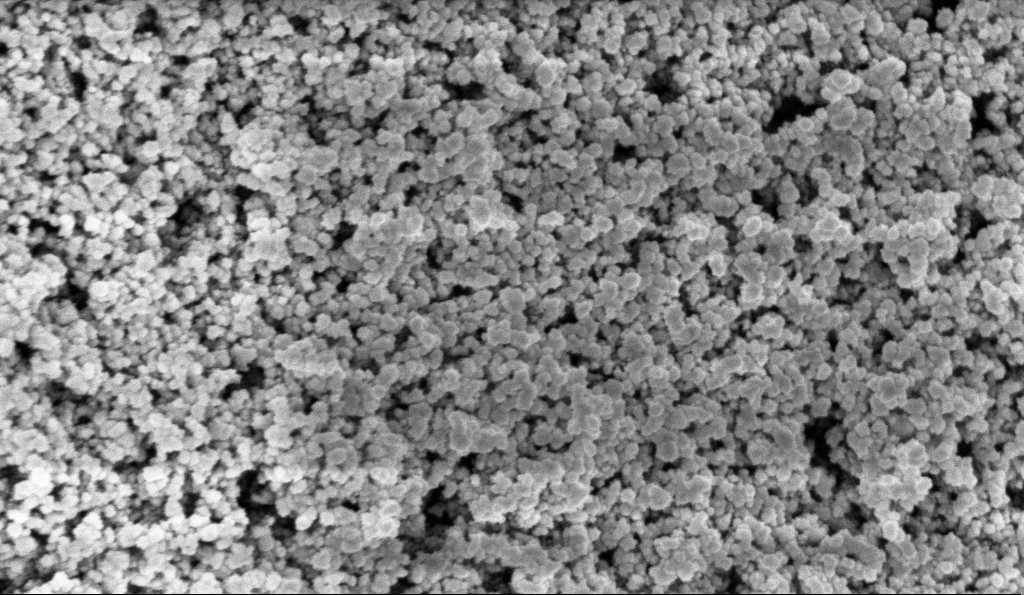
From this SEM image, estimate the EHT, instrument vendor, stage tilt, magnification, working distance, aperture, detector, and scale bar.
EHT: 3 kV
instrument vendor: Zeiss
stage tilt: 0°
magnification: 135 K X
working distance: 6 mm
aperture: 30 µm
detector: InLens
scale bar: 100 nm